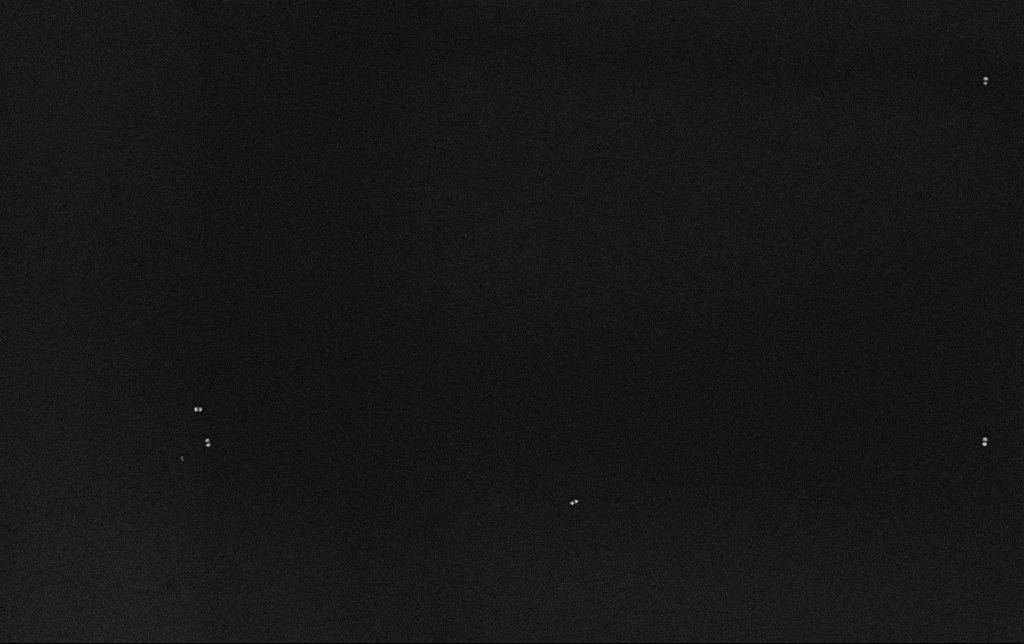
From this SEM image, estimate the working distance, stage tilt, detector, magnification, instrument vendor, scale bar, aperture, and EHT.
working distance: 3.2 mm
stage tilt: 0°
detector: InLens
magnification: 100 K X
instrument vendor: Zeiss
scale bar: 200 nm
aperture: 30 µm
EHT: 10 kV